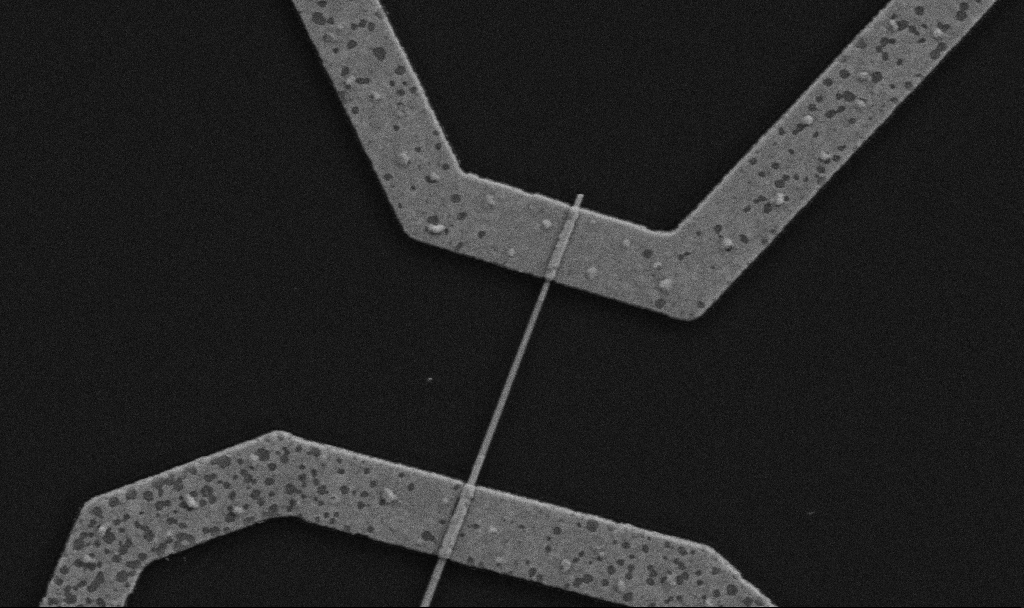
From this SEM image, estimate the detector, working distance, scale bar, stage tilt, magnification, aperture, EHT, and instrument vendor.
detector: SE2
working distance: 10.7 mm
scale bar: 1000 nm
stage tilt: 0°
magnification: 30 K X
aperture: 30 µm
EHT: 5 kV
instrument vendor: Zeiss